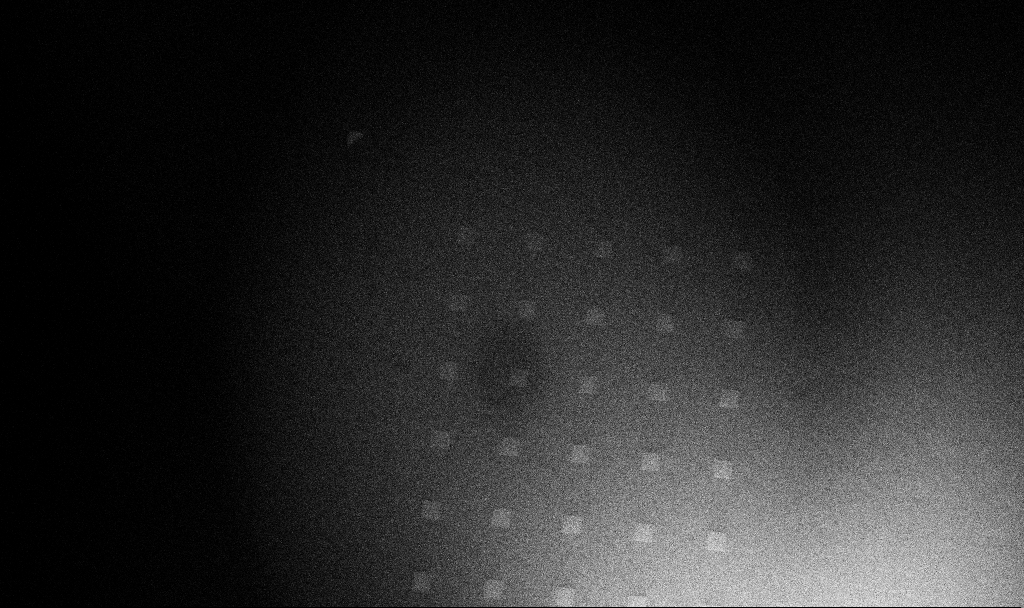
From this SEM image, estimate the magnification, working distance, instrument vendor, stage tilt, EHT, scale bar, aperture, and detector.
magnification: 0.067 K X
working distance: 9.1 mm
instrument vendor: Zeiss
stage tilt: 45°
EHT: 5 kV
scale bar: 1e+06 nm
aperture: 30 µm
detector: InLens